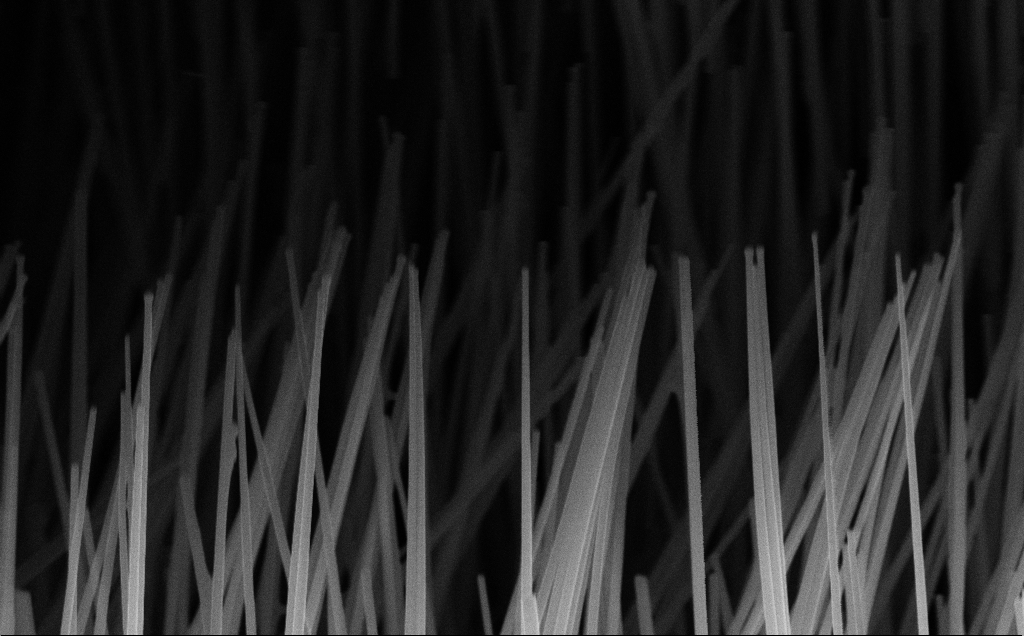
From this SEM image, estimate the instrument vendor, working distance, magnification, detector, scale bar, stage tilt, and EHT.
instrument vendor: Zeiss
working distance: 6 mm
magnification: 39.45 K X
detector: InLens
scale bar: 1000 nm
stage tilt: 44.7°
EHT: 10 kV